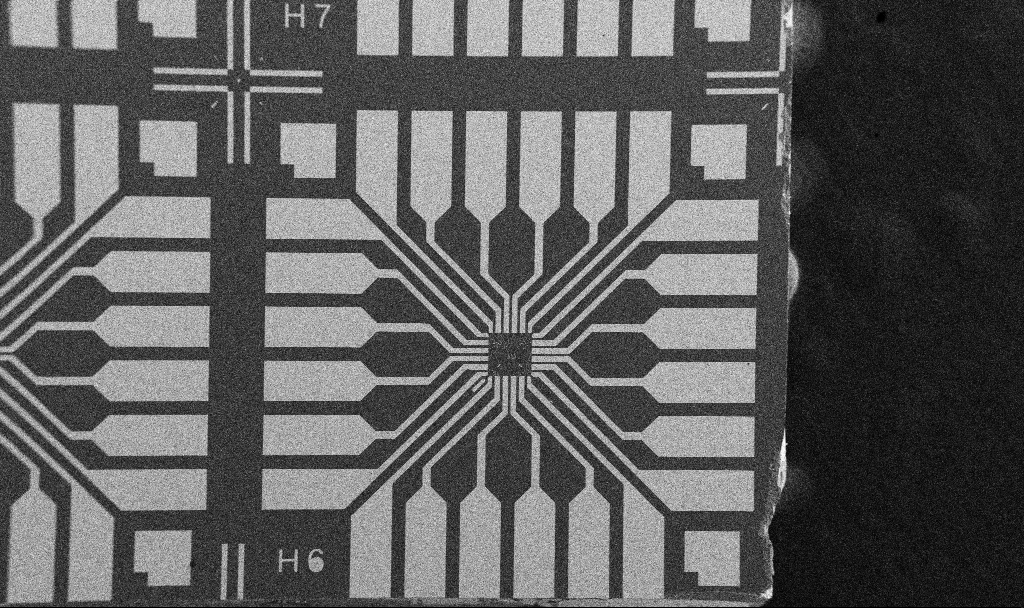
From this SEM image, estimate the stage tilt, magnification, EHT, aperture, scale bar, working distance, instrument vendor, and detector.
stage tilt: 0°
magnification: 0.1 K X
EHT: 5 kV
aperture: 30 µm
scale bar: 200000 nm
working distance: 10.7 mm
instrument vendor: Zeiss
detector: SE2